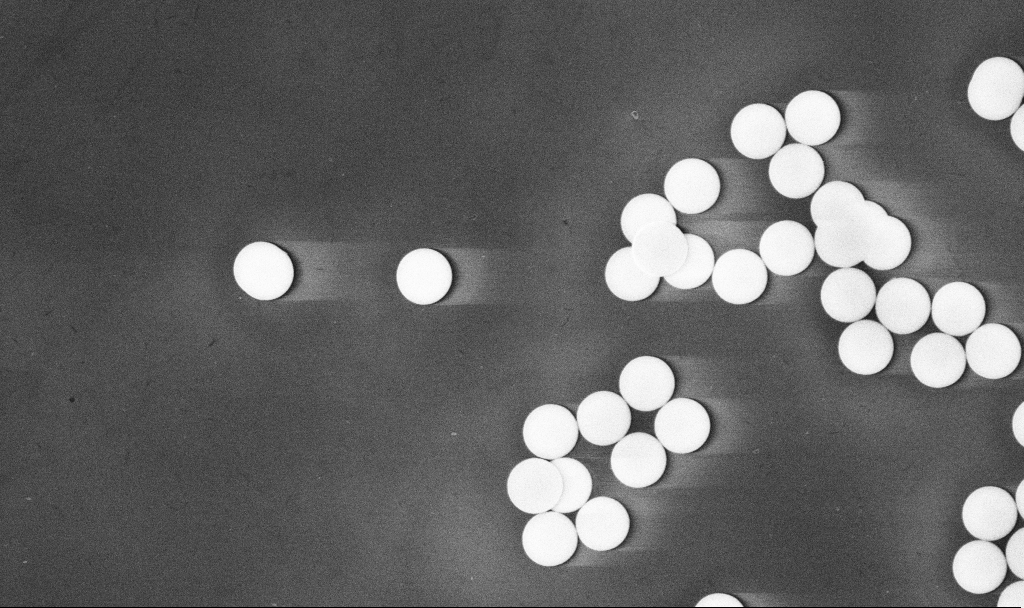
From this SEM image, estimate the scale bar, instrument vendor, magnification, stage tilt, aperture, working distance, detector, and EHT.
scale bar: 2000 nm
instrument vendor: Zeiss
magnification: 15.84 K X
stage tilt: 0°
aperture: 30 µm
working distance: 5.4 mm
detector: InLens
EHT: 10 kV